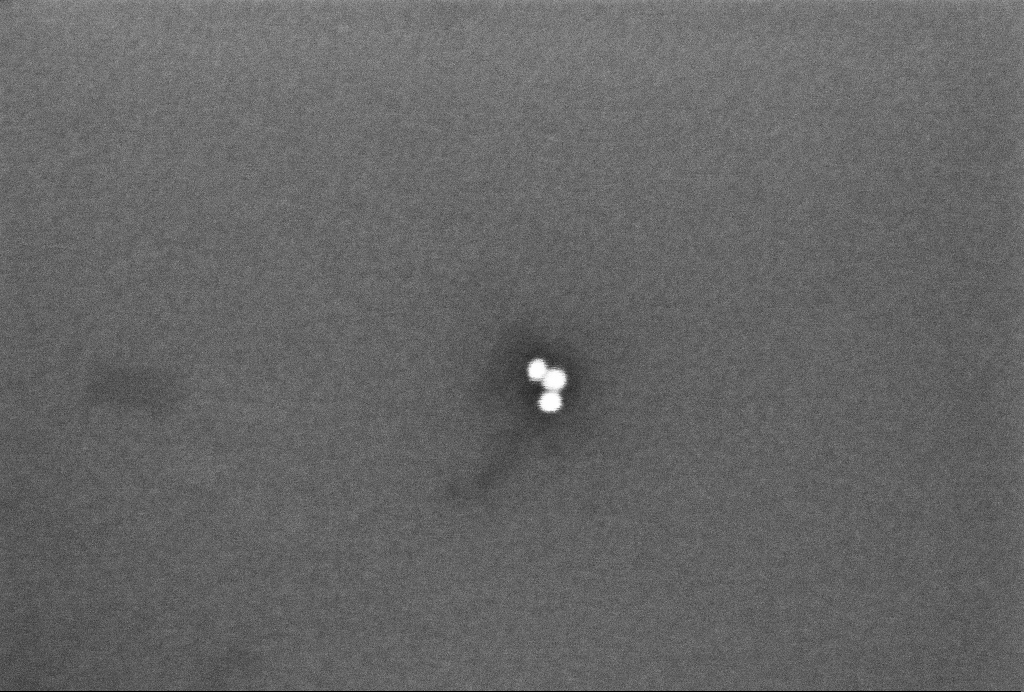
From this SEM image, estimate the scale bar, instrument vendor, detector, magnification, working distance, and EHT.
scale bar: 200 nm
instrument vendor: Zeiss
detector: InLens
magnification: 287.22 K X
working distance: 3.3 mm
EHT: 2 kV